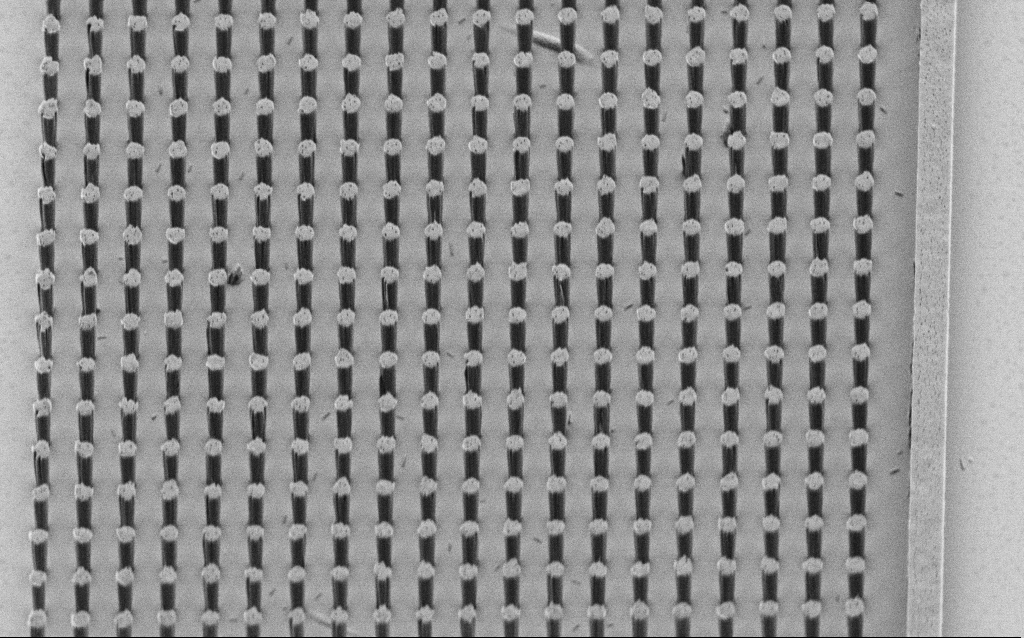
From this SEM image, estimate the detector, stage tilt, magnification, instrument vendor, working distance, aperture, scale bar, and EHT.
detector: SE2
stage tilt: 45°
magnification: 13.03 K X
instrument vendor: Zeiss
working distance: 7 mm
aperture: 30 µm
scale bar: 2000 nm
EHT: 1.5 kV